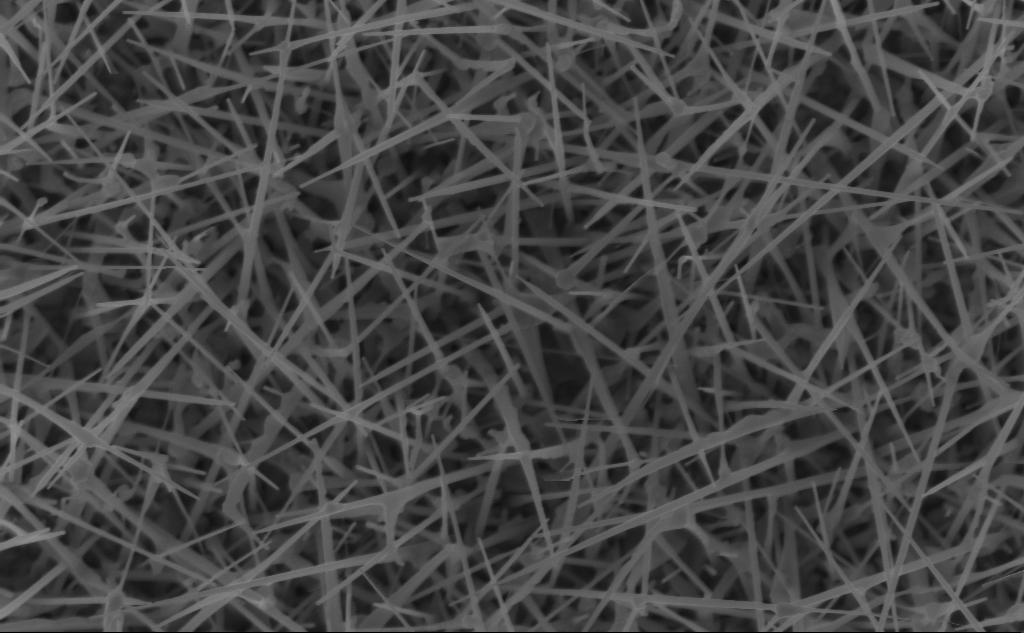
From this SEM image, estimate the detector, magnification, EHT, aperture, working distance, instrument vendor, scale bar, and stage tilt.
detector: InLens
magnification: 40 K X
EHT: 5 kV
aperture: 30 µm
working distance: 4 mm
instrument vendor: Zeiss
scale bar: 1000 nm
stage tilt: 0°